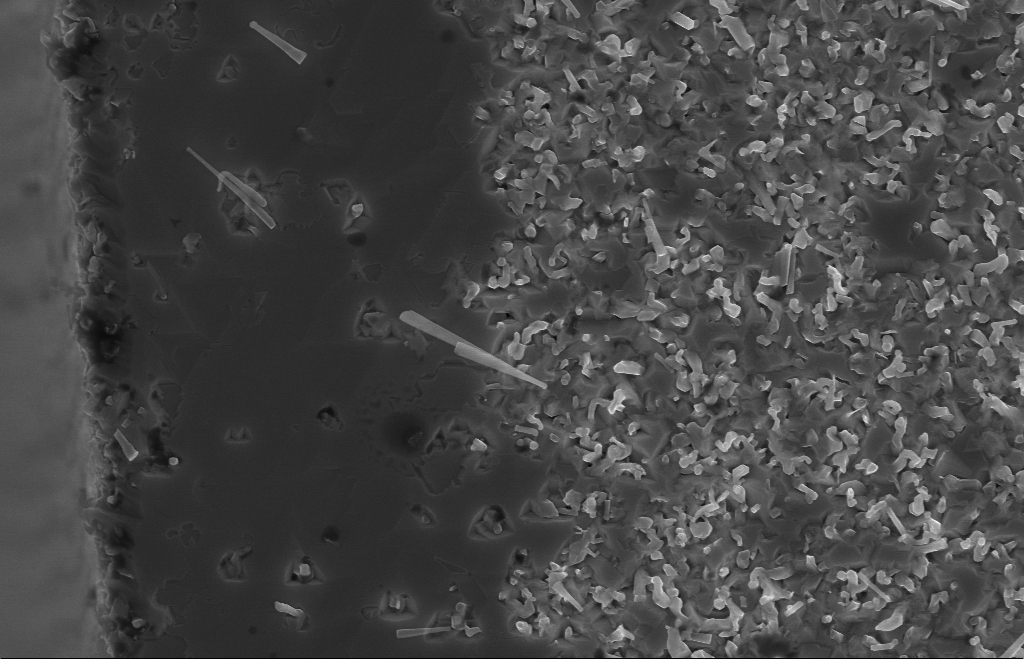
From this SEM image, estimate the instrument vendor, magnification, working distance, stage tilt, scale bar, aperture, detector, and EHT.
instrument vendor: Zeiss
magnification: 20 K X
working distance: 9 mm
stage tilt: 0°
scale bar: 1000 nm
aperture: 30 µm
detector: InLens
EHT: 10 kV